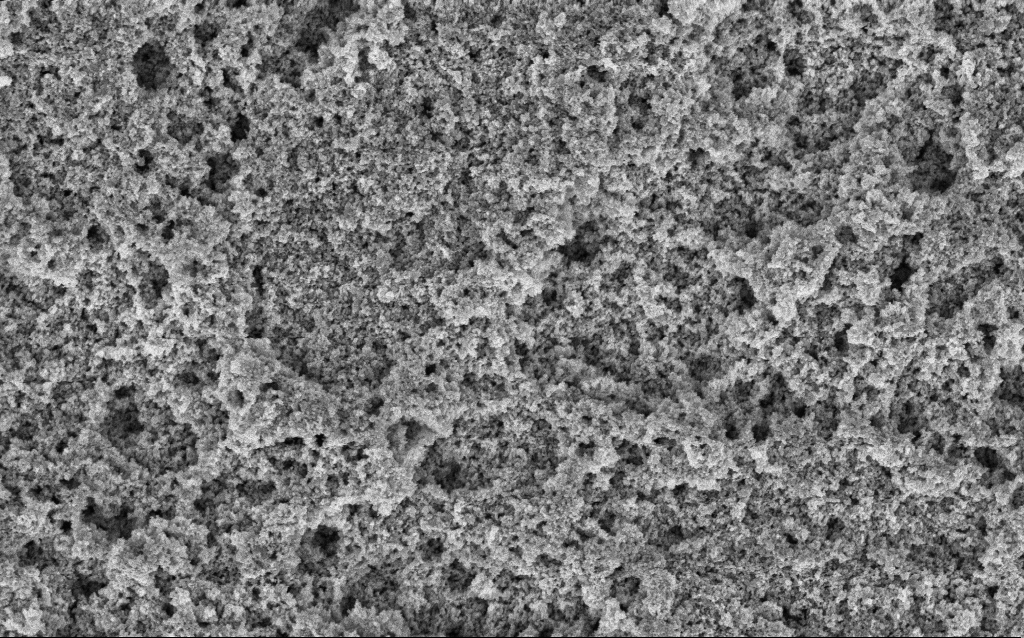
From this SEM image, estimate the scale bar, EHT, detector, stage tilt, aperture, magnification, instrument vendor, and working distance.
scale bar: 1000 nm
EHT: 3 kV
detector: InLens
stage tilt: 0°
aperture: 30 µm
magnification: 37.88 K X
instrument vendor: Zeiss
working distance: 10 mm